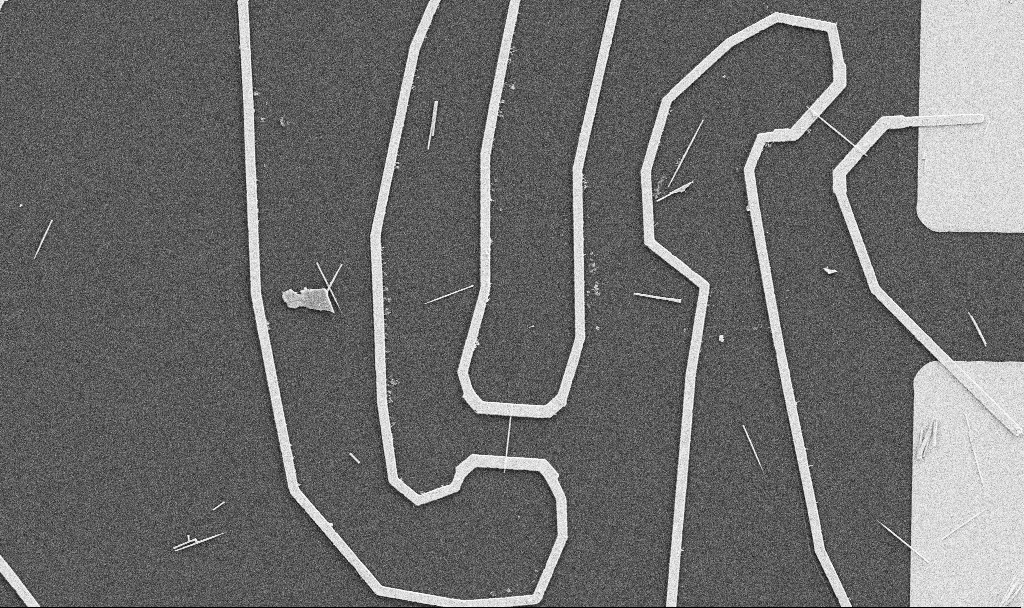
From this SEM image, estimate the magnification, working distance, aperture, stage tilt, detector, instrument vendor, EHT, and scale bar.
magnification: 5 K X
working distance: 10.7 mm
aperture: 30 µm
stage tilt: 0°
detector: SE2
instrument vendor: Zeiss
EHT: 5 kV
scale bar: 10000 nm